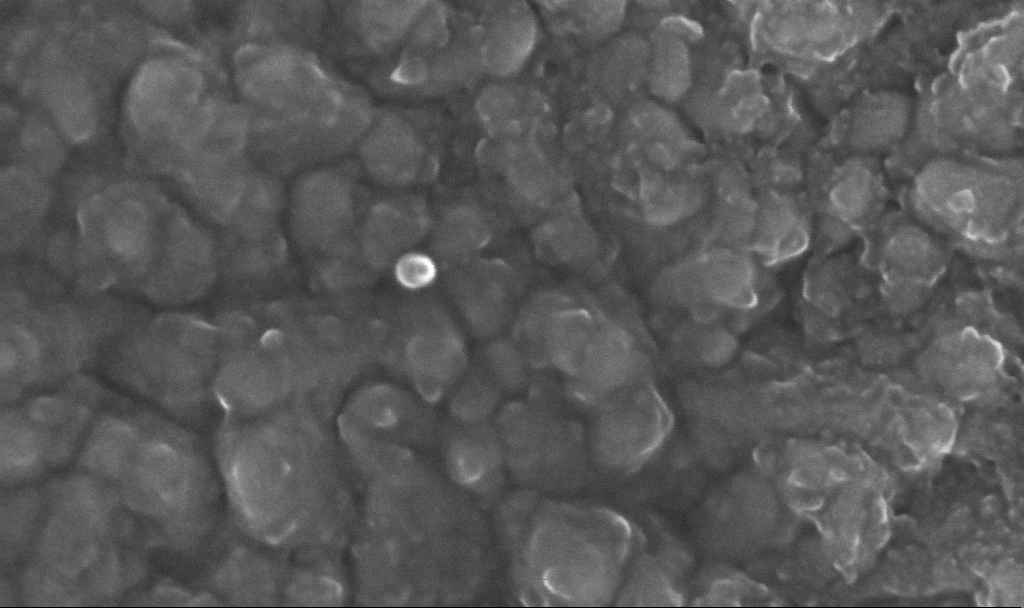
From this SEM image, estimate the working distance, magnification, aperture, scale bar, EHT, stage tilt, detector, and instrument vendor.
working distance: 4.8 mm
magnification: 400.61 K X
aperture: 30 µm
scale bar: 100 nm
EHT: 20 kV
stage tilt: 0°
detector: InLens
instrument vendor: Zeiss